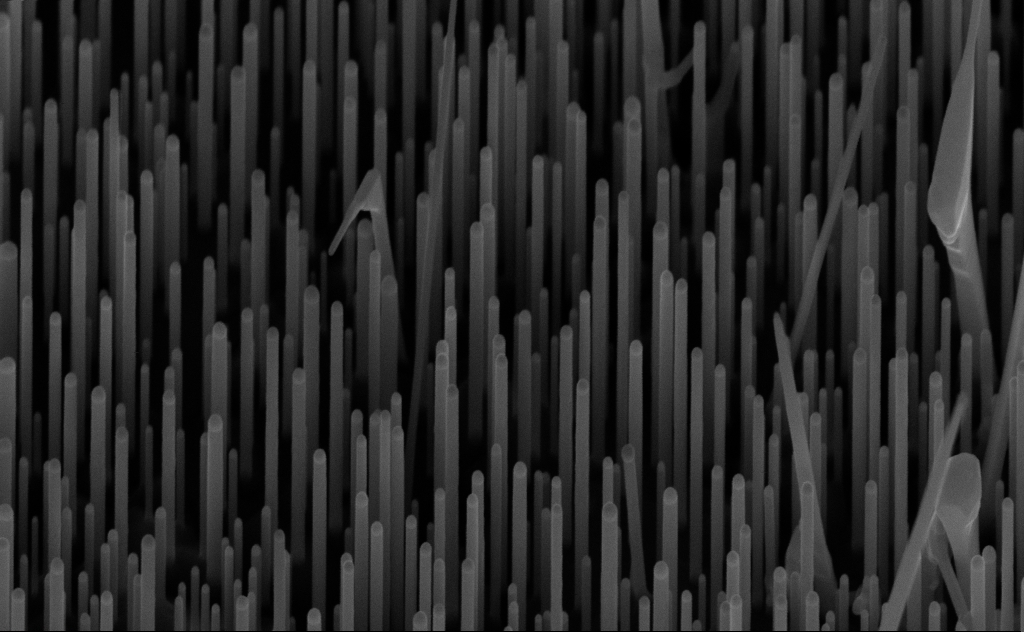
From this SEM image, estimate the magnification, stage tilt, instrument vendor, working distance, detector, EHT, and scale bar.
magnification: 80.38 K X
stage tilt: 45°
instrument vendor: Zeiss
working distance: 7 mm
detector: InLens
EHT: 10 kV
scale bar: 200 nm